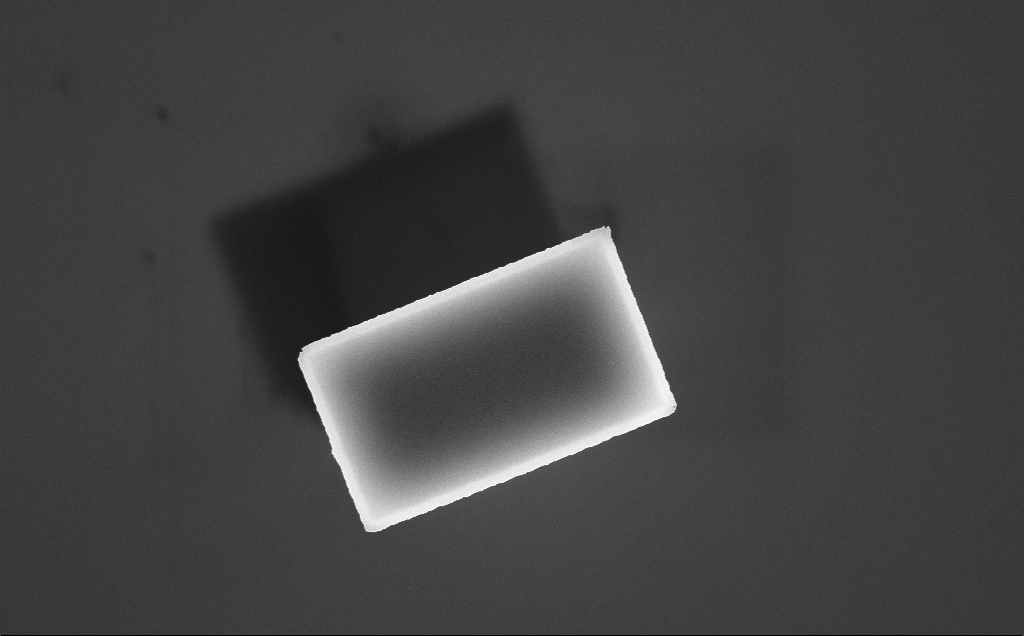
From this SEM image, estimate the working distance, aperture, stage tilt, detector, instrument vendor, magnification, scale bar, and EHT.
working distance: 3 mm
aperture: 30 µm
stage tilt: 0°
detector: InLens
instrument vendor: Zeiss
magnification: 25.62 K X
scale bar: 1000 nm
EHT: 10 kV